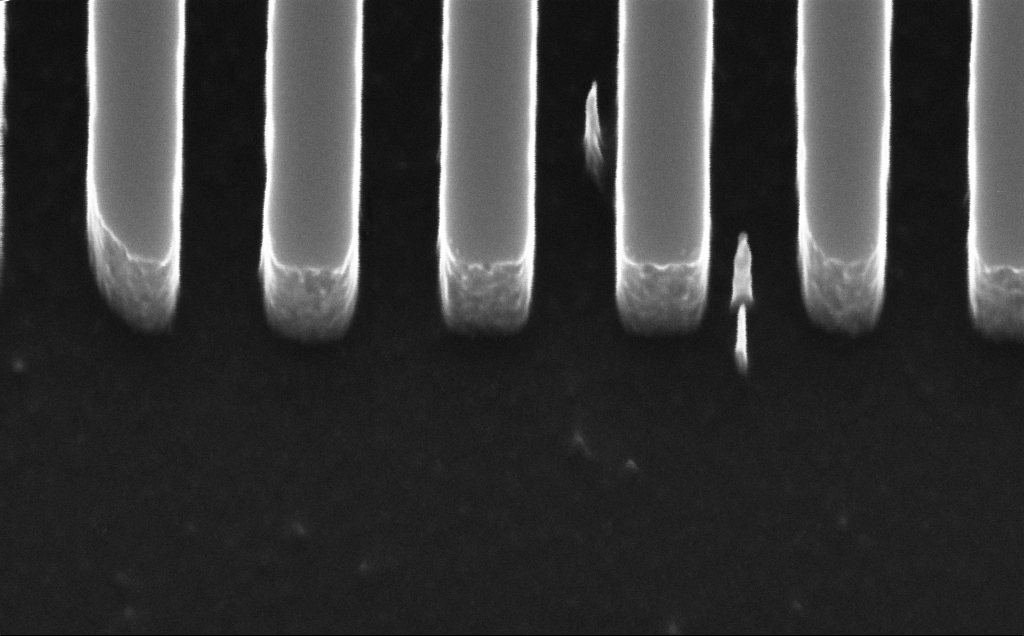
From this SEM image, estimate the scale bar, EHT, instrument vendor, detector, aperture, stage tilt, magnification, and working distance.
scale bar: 200 nm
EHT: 10 kV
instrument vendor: Zeiss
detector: InLens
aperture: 30 µm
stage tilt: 30°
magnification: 186.2 K X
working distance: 5 mm